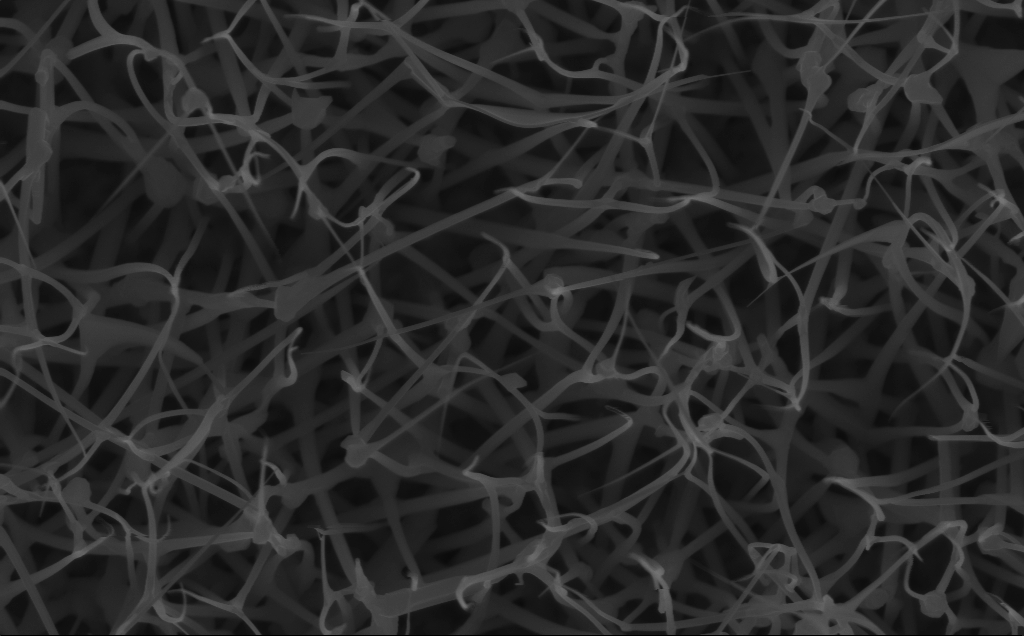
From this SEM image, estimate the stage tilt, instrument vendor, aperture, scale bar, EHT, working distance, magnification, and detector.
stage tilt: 0°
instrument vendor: Zeiss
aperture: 30 µm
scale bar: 1000 nm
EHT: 10 kV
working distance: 6 mm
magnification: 40 K X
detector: InLens